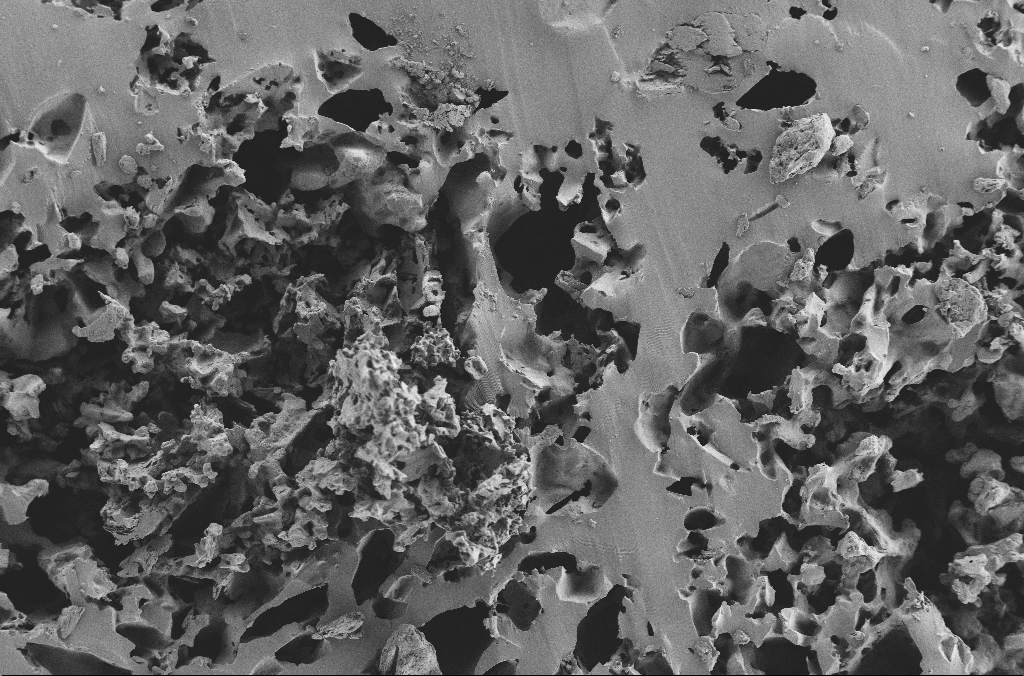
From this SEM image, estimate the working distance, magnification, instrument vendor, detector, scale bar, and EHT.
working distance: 3.1 mm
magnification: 0.25 K X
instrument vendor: Zeiss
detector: SE2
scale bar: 100000 nm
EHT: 2 kV